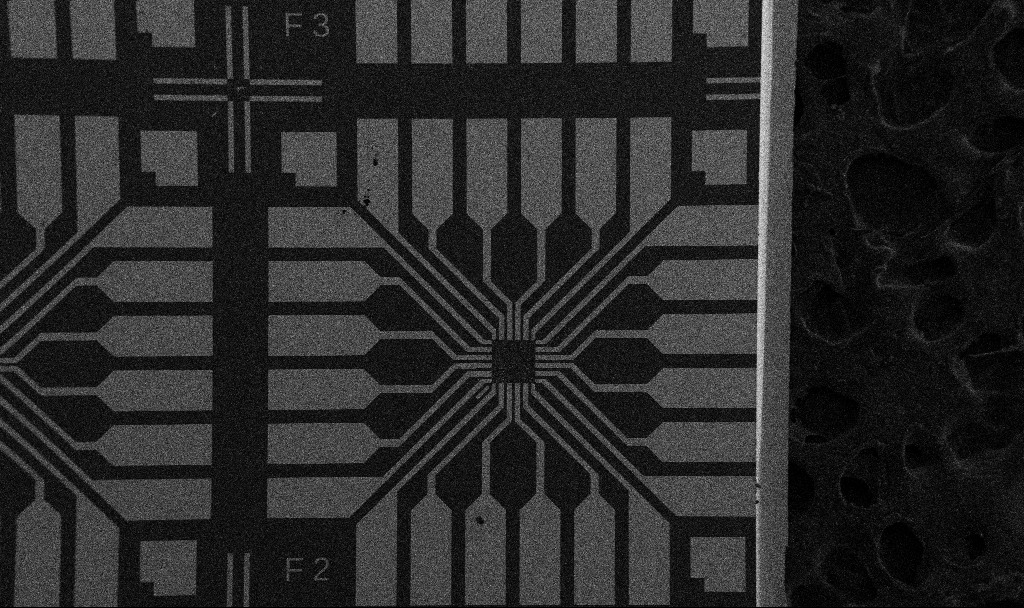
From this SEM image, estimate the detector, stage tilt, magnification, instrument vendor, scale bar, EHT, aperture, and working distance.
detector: SE2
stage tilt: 0°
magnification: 0.1 K X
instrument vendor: Zeiss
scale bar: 200000 nm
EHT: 5 kV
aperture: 30 µm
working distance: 10.7 mm